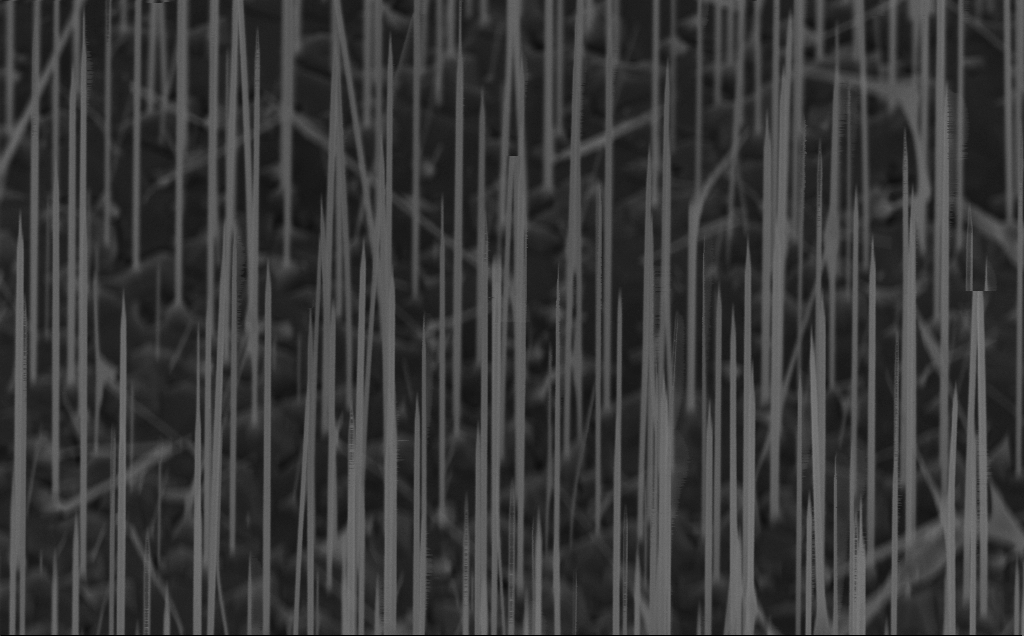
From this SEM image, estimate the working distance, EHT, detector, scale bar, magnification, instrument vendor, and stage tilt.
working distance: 8 mm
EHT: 5 kV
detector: InLens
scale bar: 1000 nm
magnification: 40 K X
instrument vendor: Zeiss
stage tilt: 45°